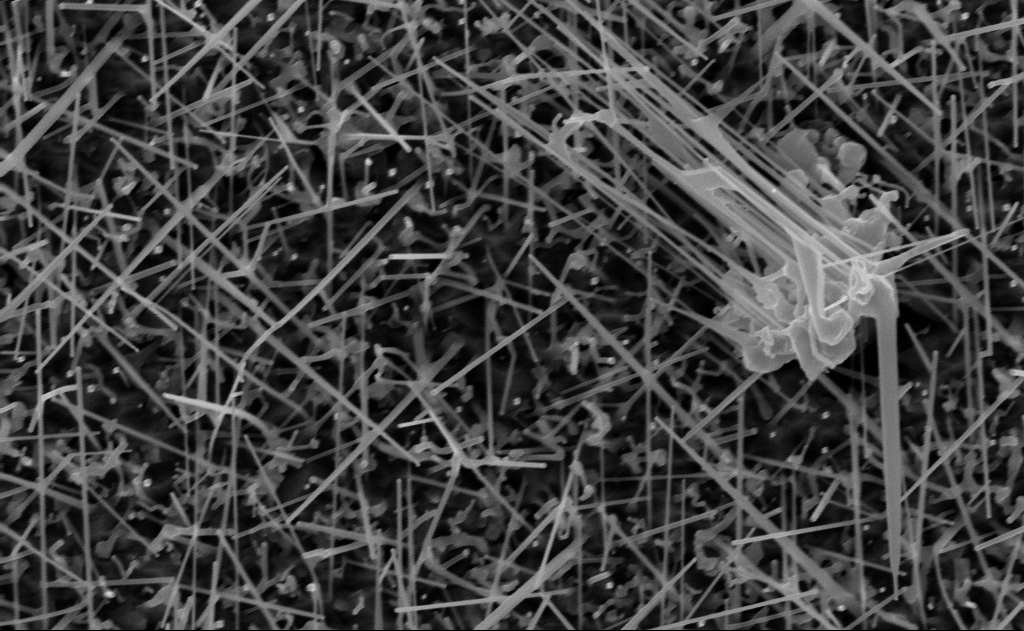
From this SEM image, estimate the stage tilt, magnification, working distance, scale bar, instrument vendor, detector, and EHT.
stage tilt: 0°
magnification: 40 K X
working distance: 15 mm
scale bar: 1000 nm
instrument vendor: Zeiss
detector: InLens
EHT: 10 kV